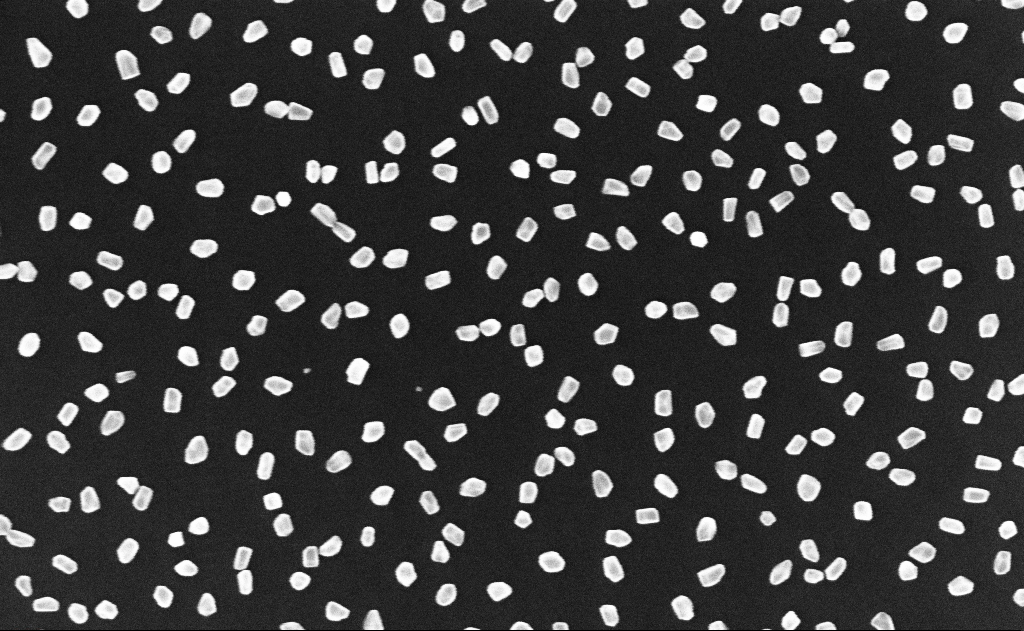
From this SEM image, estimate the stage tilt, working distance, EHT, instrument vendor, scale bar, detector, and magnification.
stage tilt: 0°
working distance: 11 mm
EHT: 10 kV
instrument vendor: Zeiss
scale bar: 200 nm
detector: InLens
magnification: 100 K X